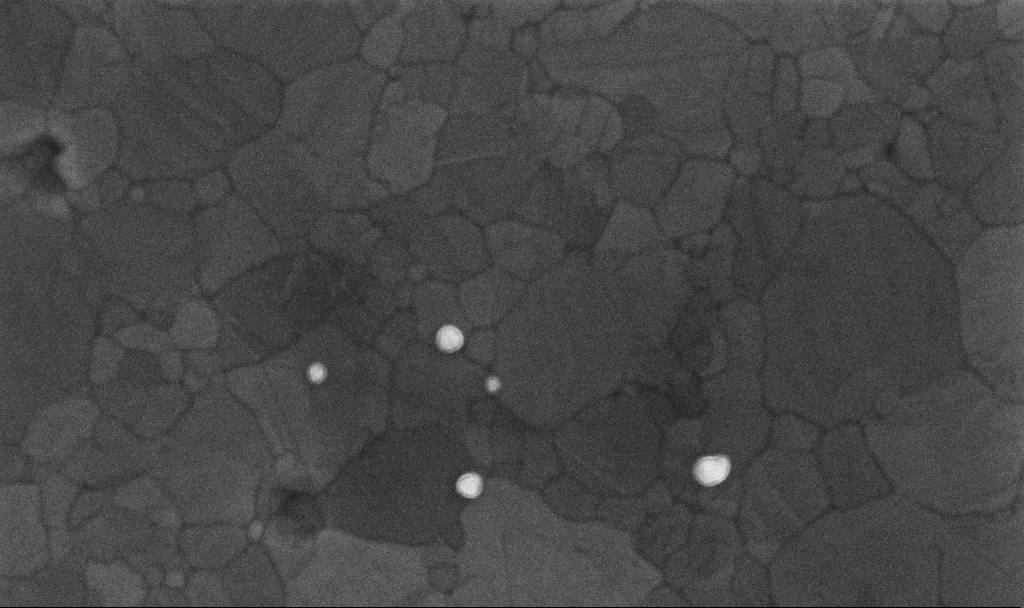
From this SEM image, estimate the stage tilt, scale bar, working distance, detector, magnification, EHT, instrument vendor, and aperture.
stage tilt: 0°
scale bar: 200 nm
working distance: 3.4 mm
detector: InLens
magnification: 181.95 K X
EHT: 10 kV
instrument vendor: Zeiss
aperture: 30 µm